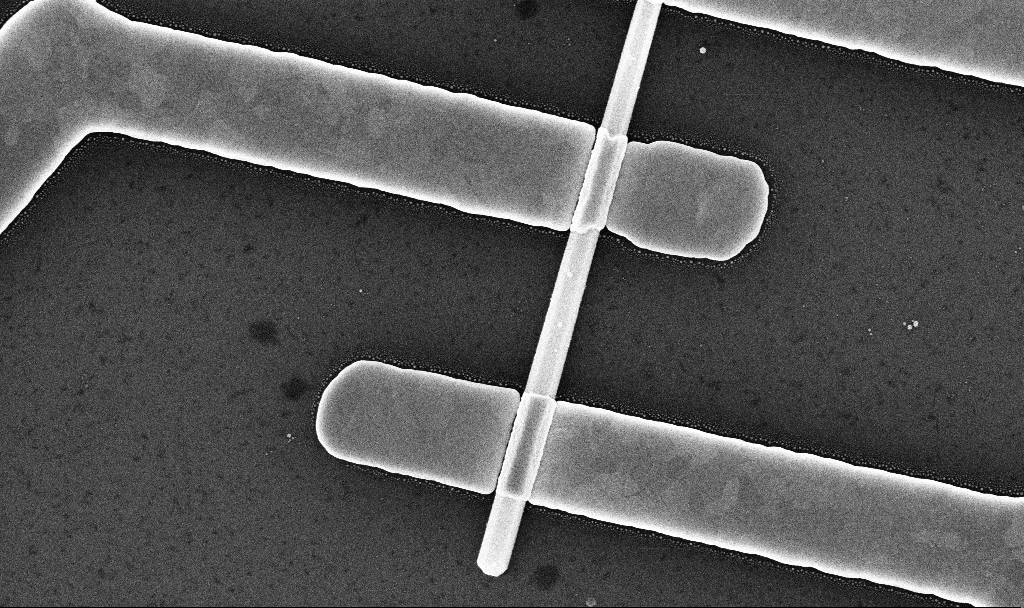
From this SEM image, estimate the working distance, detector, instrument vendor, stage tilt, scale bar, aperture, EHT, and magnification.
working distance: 7.8 mm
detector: InLens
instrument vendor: Zeiss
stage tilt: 0°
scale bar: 1000 nm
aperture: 30 µm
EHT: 10 kV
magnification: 54.57 K X